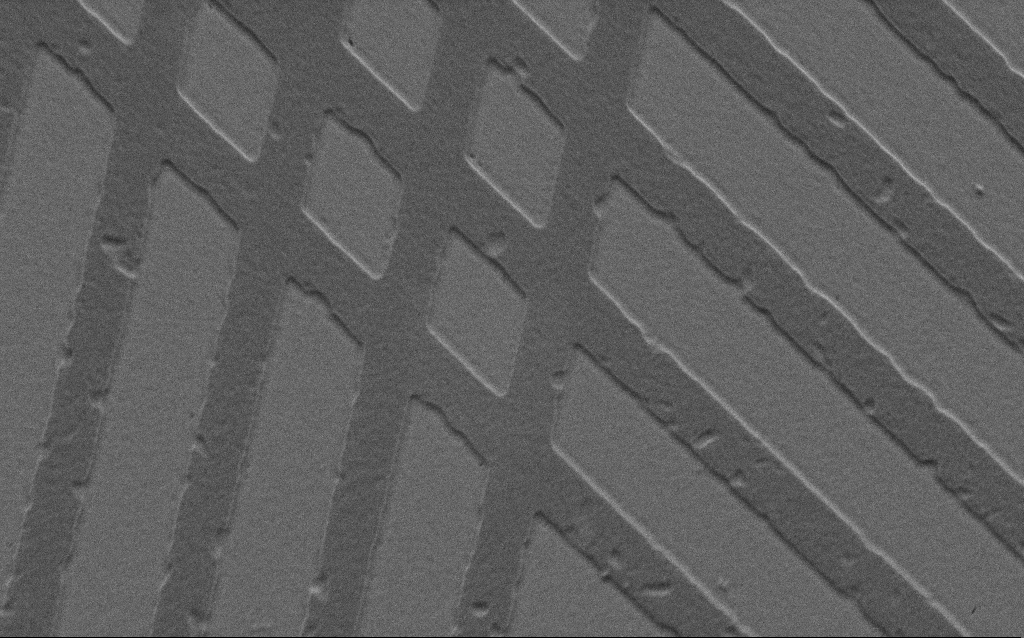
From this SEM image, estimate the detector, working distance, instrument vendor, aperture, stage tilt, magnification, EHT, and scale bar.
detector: SE2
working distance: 4 mm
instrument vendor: Zeiss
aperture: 30 µm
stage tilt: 45°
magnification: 21.95 K X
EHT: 2 kV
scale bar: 1000 nm